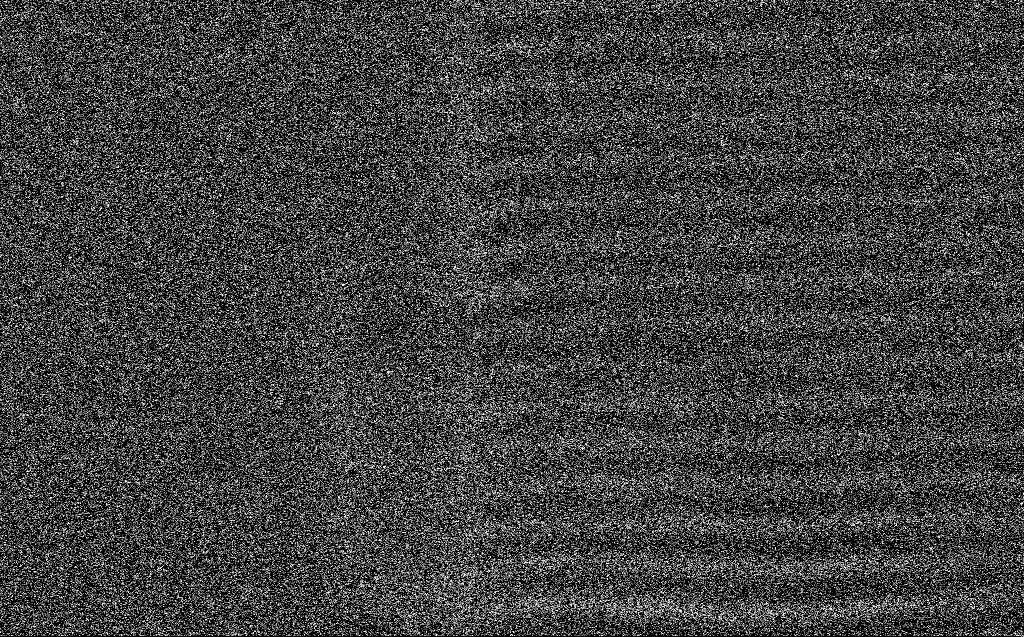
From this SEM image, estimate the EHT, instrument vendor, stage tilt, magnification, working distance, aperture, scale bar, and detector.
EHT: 5 kV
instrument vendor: Zeiss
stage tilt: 0°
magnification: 37.12 K X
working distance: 3 mm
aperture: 30 µm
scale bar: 1000 nm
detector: SE2